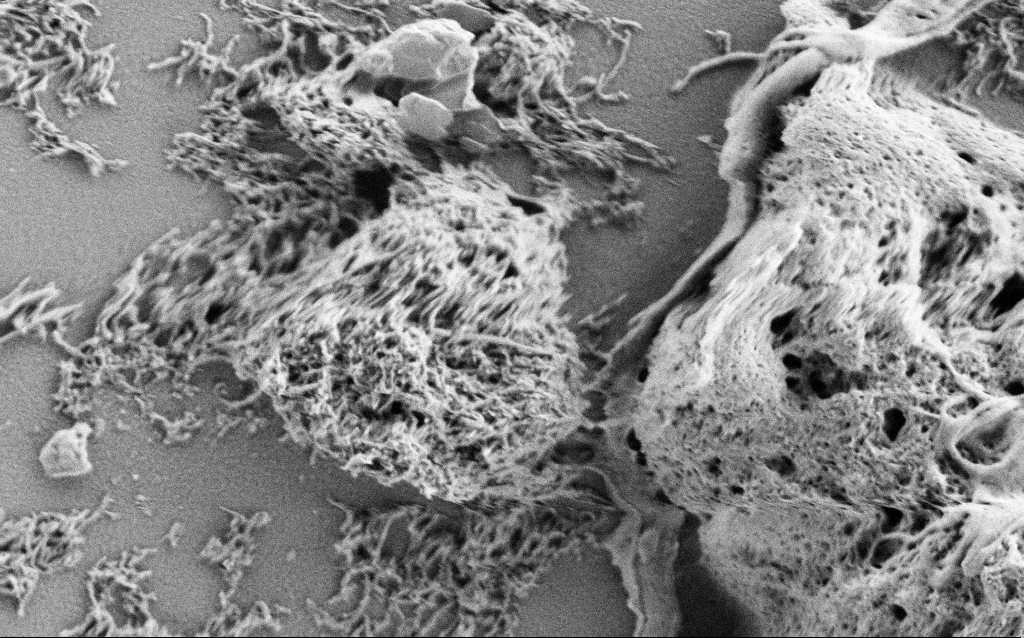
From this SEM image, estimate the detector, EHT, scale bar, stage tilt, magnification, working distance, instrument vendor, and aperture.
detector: SE2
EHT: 1 kV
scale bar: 1000 nm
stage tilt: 0°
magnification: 30 K X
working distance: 3 mm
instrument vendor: Zeiss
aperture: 30 µm